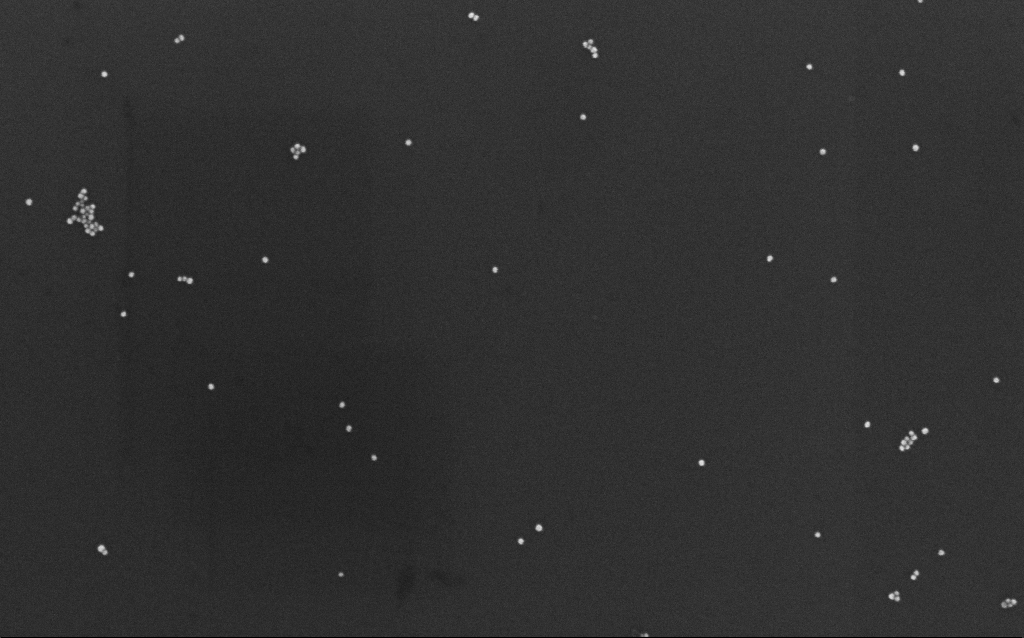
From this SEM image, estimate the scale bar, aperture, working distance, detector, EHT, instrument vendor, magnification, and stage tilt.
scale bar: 200 nm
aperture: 30 µm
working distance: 7 mm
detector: InLens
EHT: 10 kV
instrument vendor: Zeiss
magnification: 117.44 K X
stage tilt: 0°